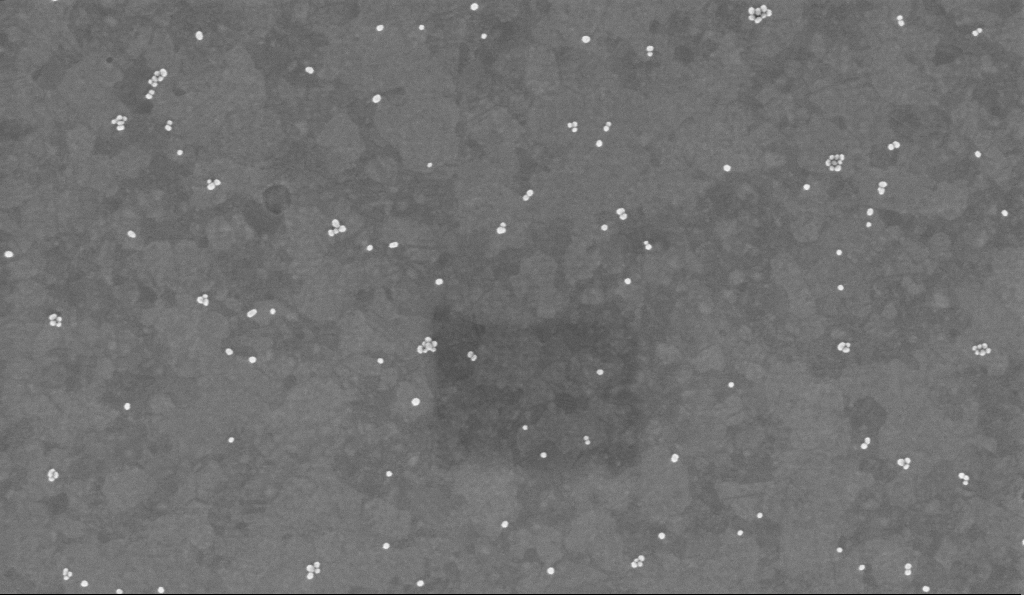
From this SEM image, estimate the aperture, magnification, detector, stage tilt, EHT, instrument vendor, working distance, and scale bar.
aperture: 30 µm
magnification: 100 K X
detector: InLens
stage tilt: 0°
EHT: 2 kV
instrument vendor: Zeiss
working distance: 3.4 mm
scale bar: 200 nm